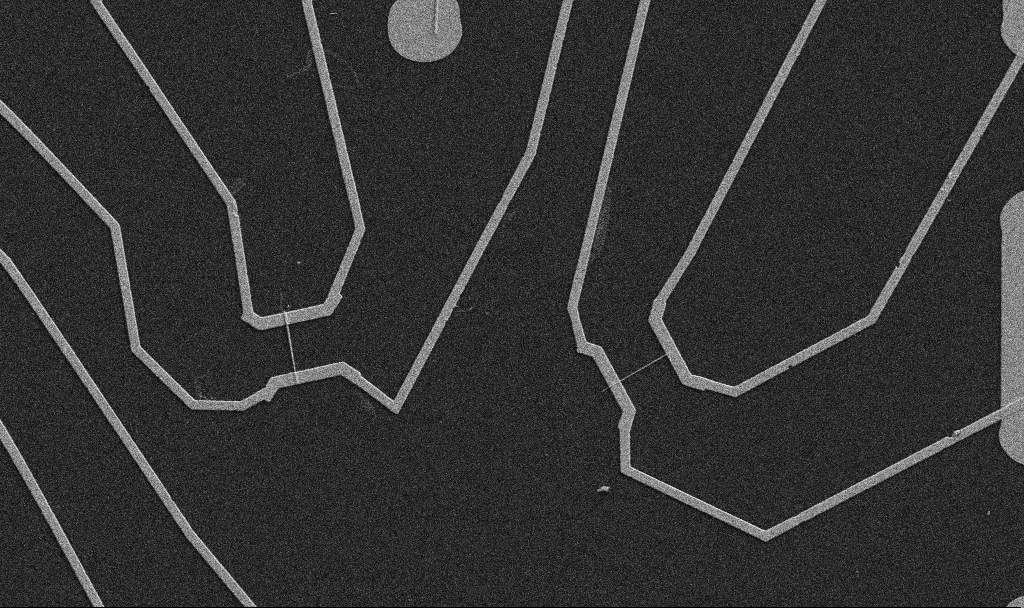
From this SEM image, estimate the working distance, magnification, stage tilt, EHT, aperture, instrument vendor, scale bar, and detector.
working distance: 10.7 mm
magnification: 5 K X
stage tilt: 0°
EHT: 5 kV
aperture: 30 µm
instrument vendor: Zeiss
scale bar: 10000 nm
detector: SE2